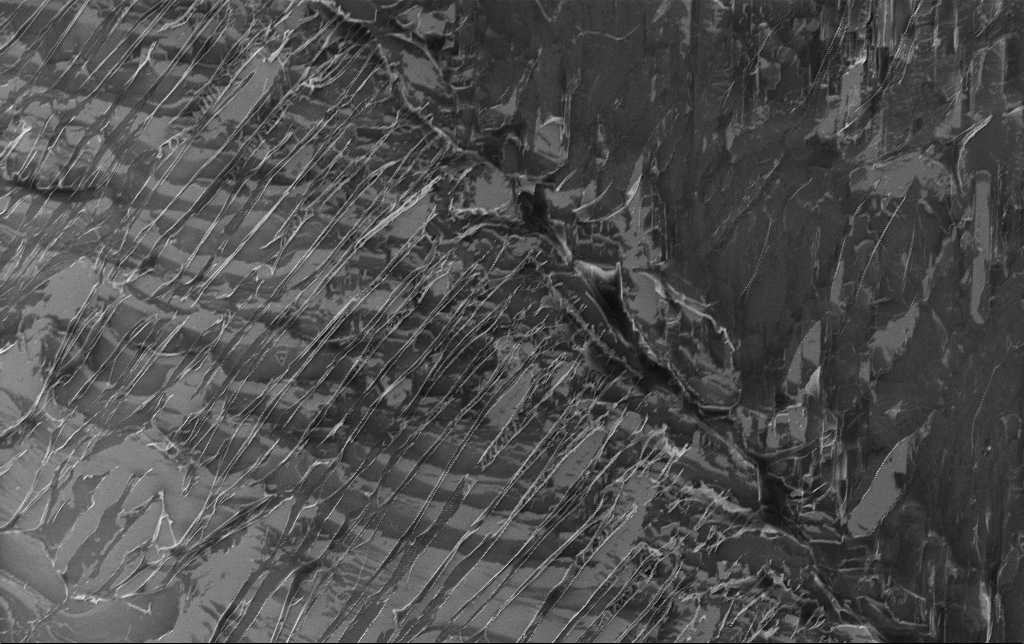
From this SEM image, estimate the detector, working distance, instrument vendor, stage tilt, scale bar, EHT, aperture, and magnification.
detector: InLens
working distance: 3.1 mm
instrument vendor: Zeiss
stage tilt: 0°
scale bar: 20000 nm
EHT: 3 kV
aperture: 30 µm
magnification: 1.28 K X